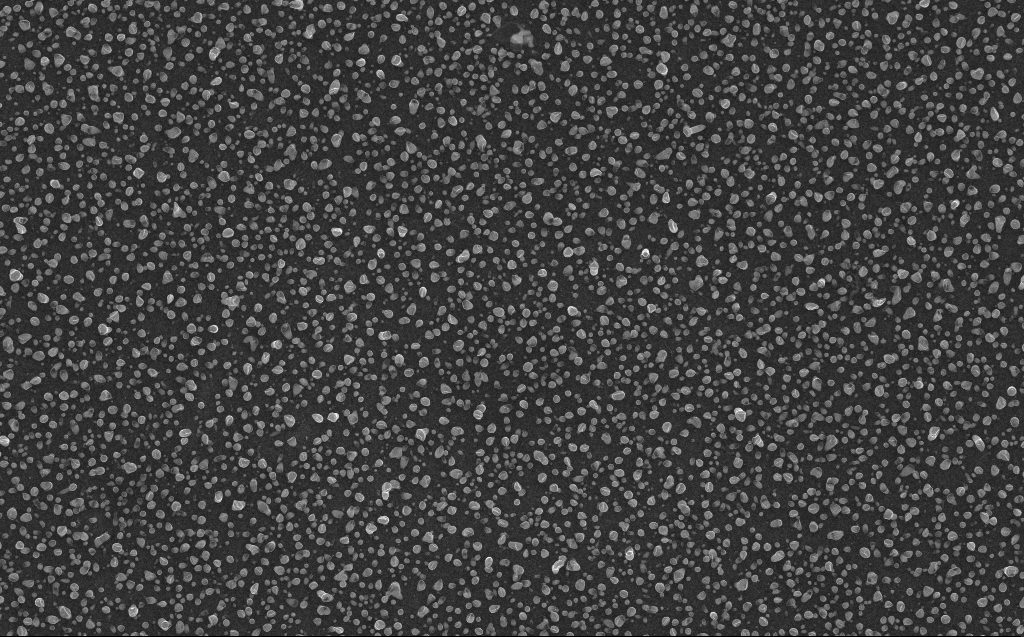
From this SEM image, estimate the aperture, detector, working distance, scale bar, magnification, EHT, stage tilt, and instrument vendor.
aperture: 30 µm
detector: InLens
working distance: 3 mm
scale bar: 2000 nm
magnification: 20 K X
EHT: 10 kV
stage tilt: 0°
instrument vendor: Zeiss